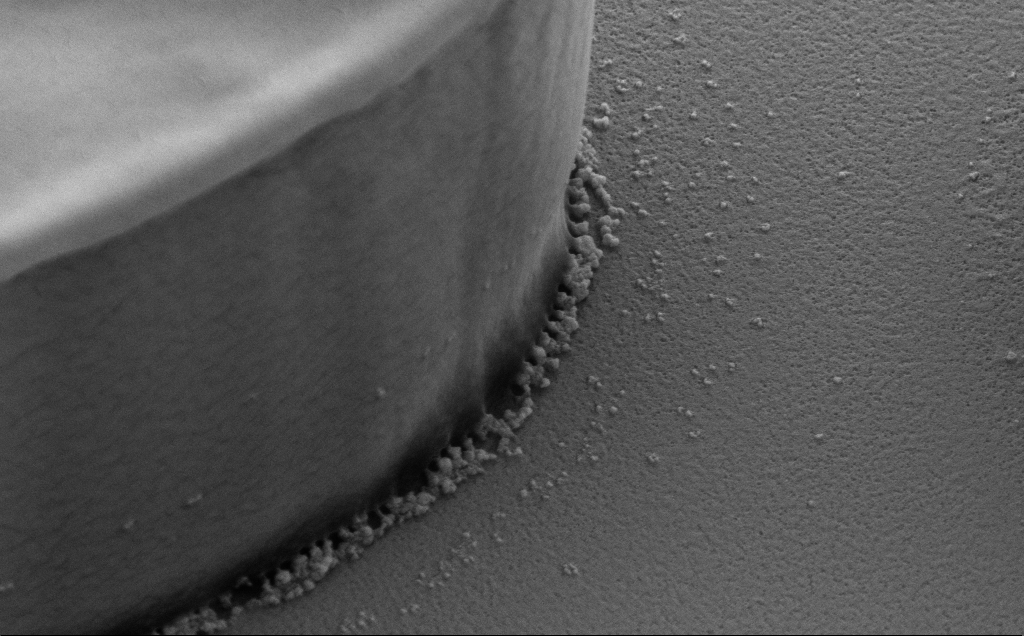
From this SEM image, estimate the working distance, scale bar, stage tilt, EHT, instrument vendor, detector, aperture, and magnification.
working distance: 8 mm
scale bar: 2000 nm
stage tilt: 40°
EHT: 5 kV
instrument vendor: Zeiss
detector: SE2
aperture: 30 µm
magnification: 36.26 K X